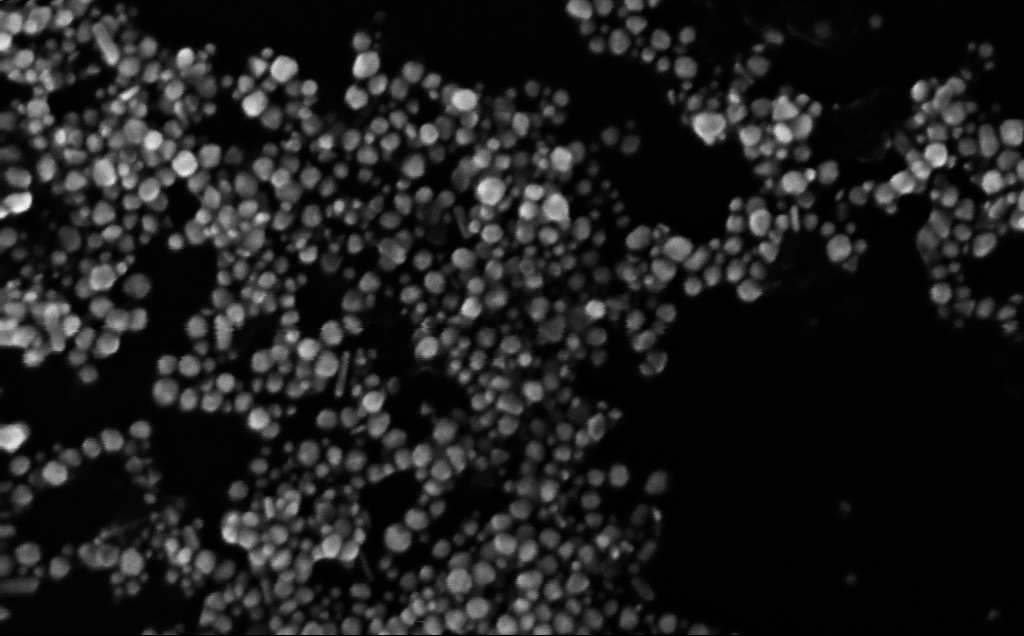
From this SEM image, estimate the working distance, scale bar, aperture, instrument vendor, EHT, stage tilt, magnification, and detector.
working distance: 4 mm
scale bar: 200 nm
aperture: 30 µm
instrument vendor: Zeiss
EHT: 10 kV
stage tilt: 0°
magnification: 342.43 K X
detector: InLens